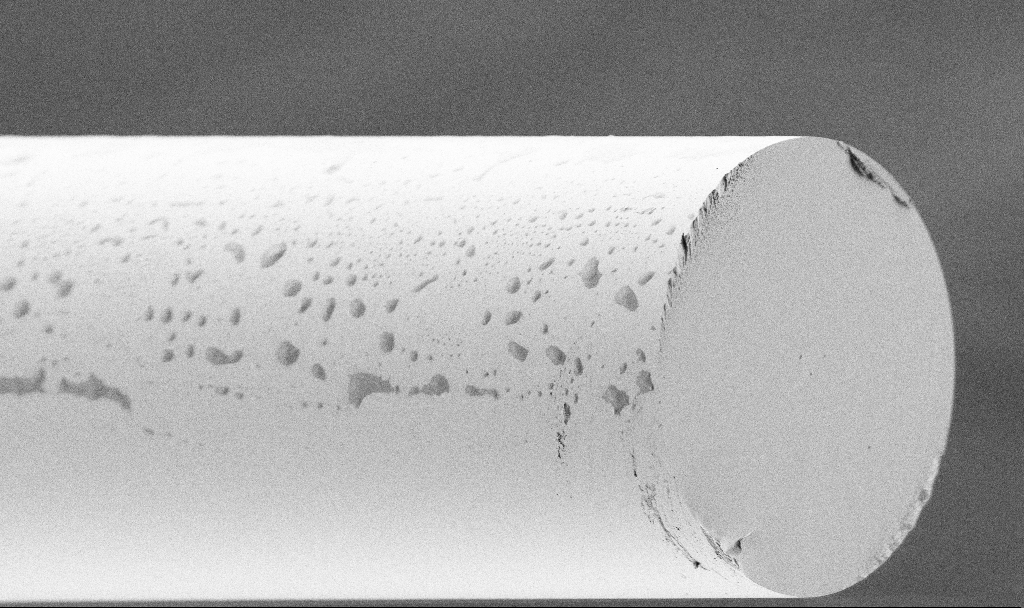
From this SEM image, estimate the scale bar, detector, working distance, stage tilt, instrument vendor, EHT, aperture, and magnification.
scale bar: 20000 nm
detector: SE2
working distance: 3.3 mm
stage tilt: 45°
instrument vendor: Zeiss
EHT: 1.5 kV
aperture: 30 µm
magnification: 1.34 K X